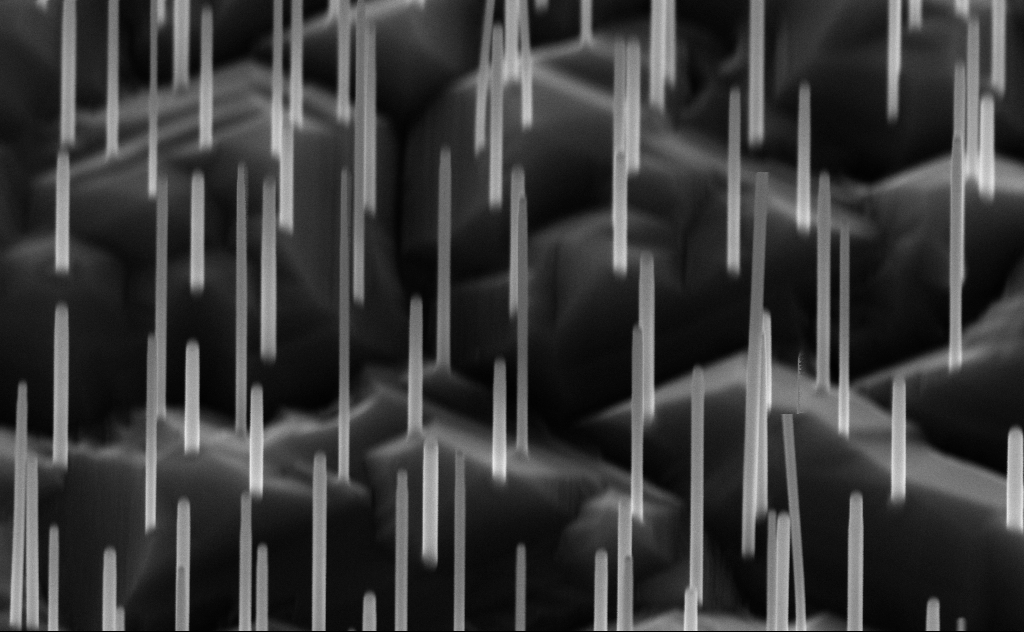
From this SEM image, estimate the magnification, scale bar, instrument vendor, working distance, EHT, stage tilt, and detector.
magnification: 80 K X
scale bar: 200 nm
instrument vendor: Zeiss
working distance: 6 mm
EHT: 10 kV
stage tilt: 44.2°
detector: InLens